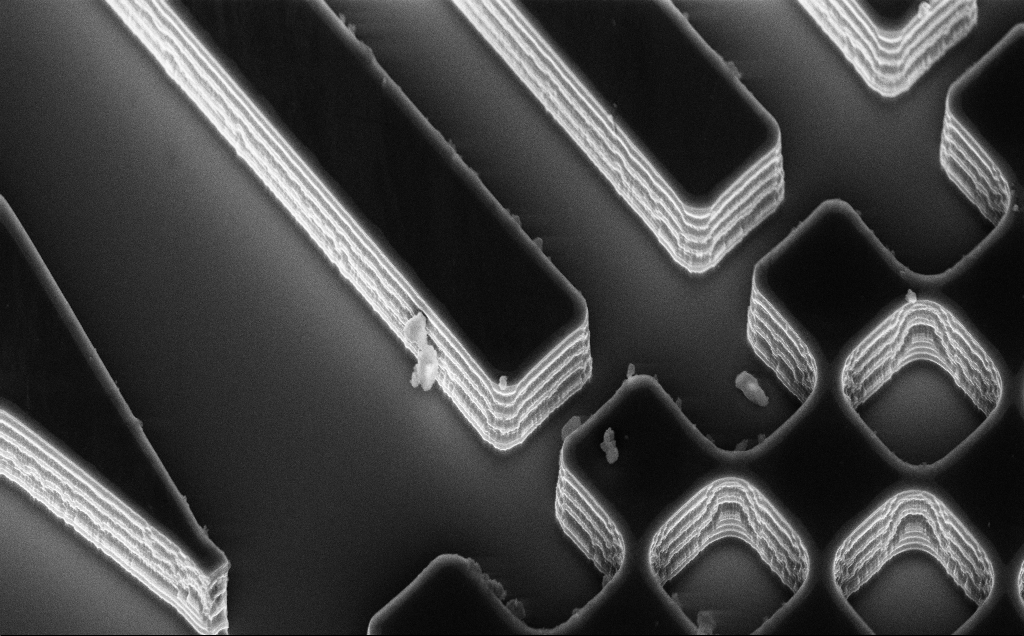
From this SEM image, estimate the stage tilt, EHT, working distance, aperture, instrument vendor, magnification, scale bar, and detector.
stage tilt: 50°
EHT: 10 kV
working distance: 10 mm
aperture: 30 µm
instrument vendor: Zeiss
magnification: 4.81 K X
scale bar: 10000 nm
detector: InLens